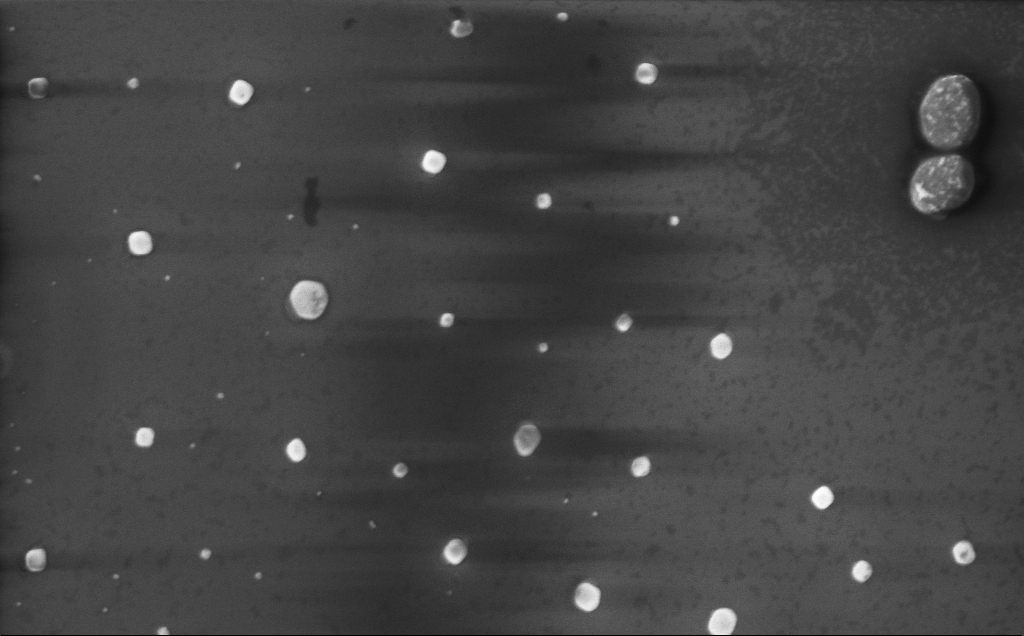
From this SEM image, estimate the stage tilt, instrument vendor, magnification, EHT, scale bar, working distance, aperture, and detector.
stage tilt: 0°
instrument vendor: Zeiss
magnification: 40 K X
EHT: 10 kV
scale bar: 1000 nm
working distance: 5 mm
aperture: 30 µm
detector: InLens